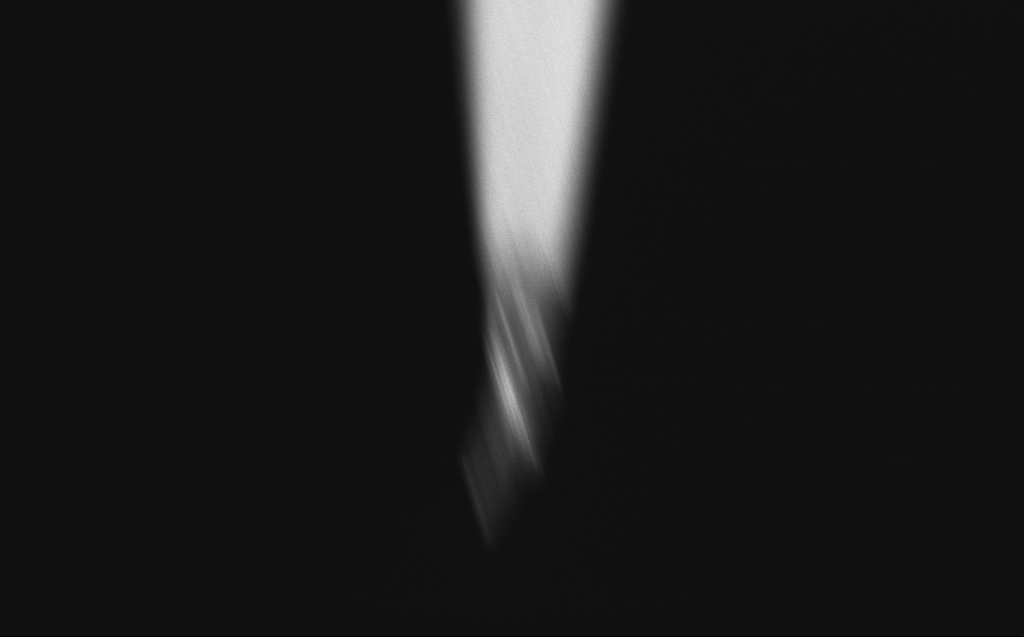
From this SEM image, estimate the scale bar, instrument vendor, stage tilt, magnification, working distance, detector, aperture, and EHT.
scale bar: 1000 nm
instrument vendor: Zeiss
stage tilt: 45°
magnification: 42.11 K X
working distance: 3 mm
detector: InLens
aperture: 20 µm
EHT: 5 kV